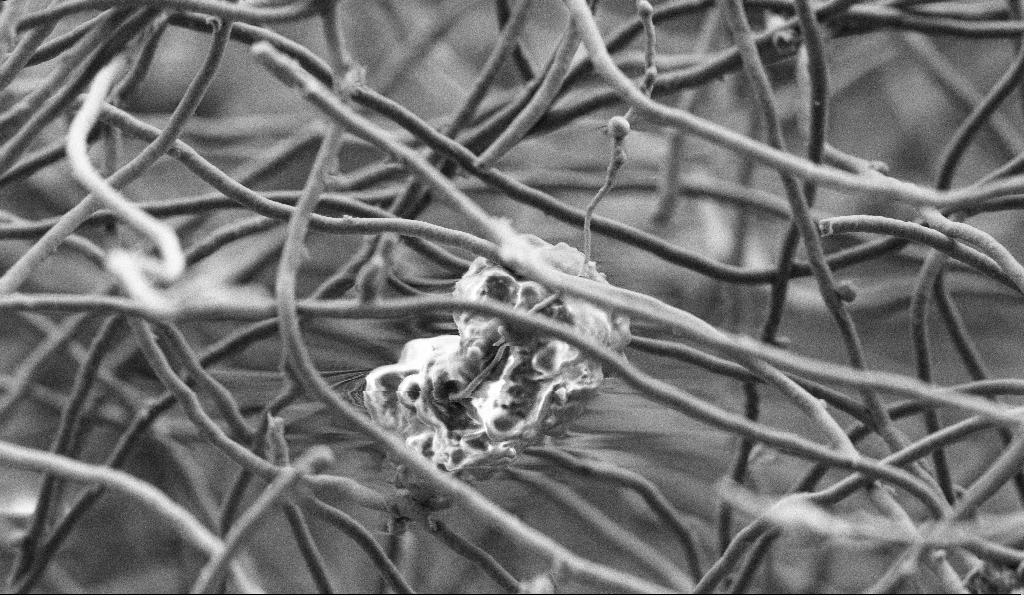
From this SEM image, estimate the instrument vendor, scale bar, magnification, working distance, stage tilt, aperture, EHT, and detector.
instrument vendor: Zeiss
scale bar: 2000 nm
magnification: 10 K X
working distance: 5 mm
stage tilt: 0°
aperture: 30 µm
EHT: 3 kV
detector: SE2